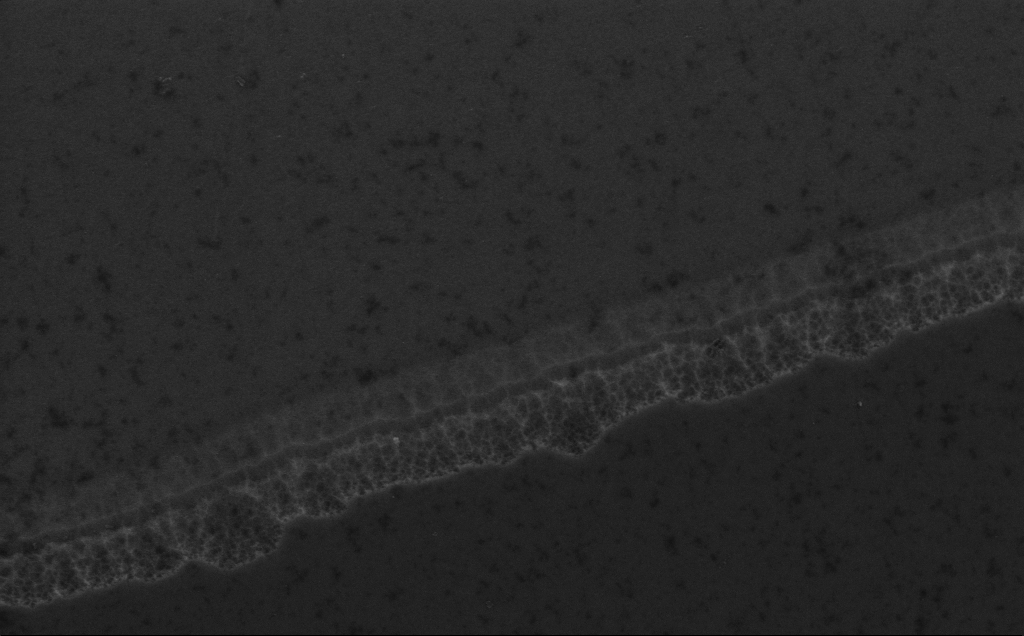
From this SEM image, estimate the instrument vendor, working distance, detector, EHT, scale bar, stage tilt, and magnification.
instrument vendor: Zeiss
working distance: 6 mm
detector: InLens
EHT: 5 kV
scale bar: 1000 nm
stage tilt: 0°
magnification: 38.48 K X